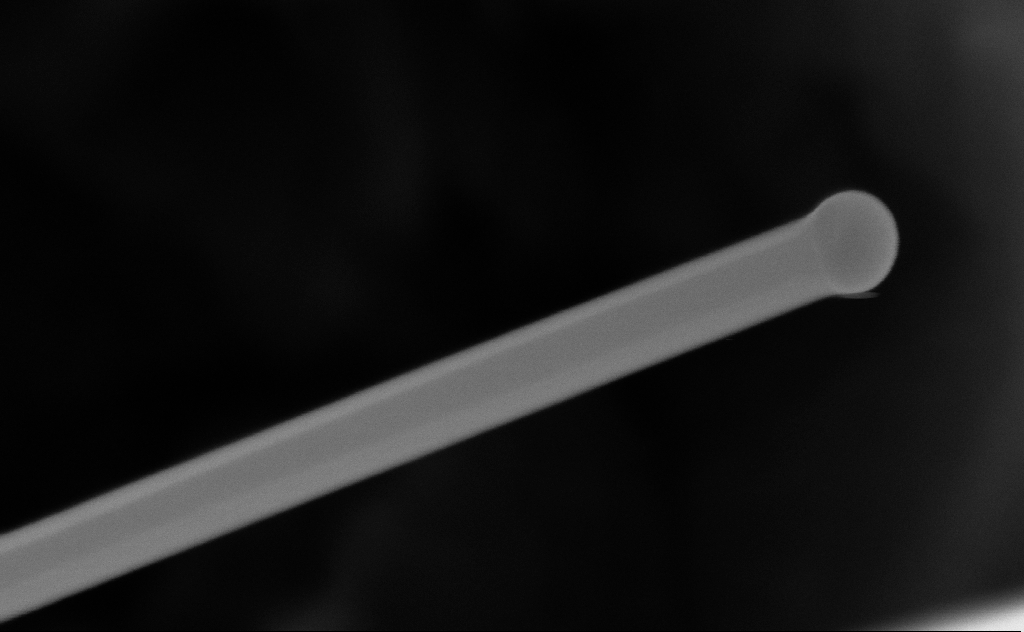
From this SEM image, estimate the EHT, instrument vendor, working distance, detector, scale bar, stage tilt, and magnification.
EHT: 10 kV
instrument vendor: Zeiss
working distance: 6 mm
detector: InLens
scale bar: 200 nm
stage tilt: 0°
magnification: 283.44 K X